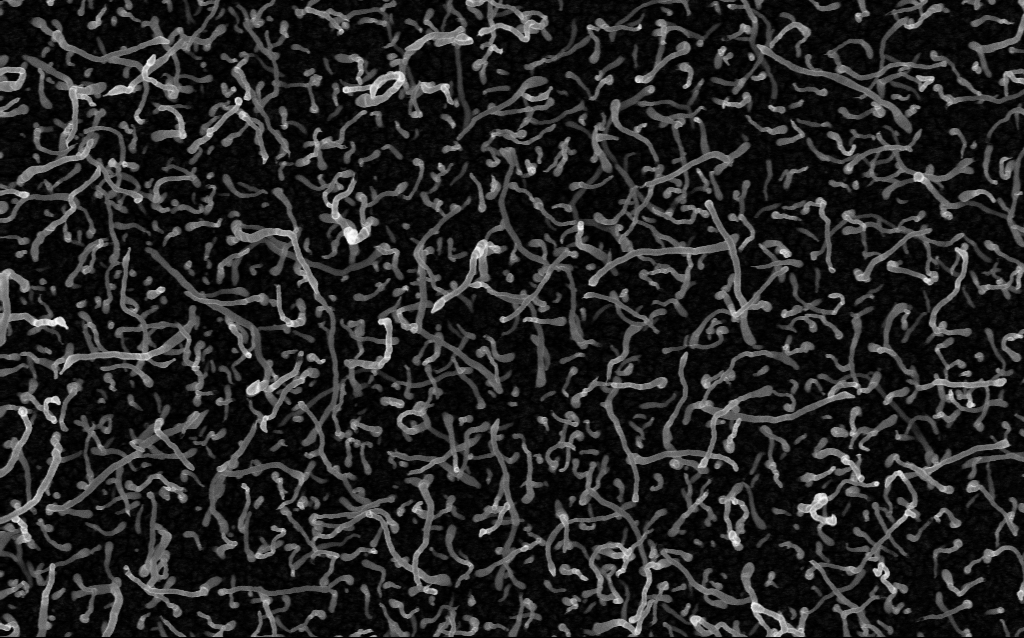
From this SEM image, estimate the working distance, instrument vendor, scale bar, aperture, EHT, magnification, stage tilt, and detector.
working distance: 2 mm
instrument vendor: Zeiss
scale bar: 1000 nm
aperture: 30 µm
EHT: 5 kV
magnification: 50 K X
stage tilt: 0°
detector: InLens